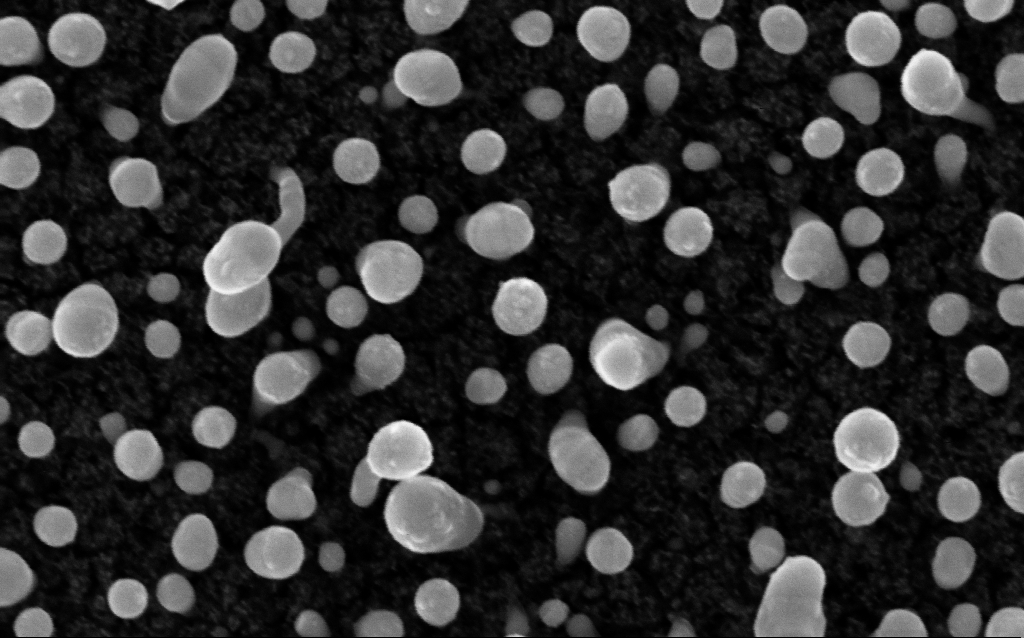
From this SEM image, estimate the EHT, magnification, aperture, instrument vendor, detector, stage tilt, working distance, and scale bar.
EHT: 5 kV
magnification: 200 K X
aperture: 30 µm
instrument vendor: Zeiss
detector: InLens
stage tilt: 0°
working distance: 2.1 mm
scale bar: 100 nm